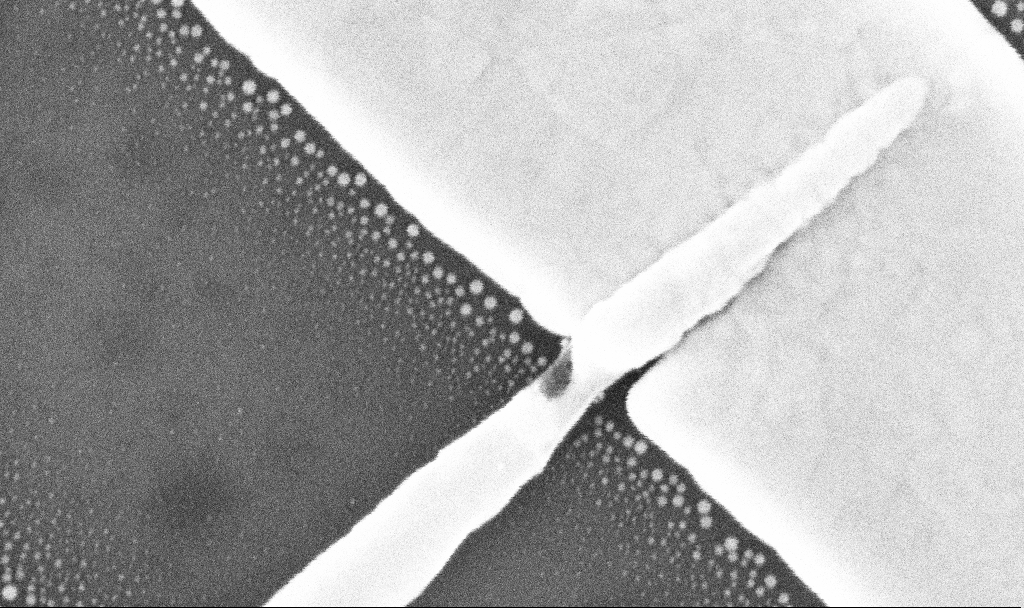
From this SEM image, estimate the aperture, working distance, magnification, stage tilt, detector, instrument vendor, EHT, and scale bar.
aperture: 30 µm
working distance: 7.7 mm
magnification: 299.84 K X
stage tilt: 0°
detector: InLens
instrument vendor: Zeiss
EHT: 10 kV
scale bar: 200 nm